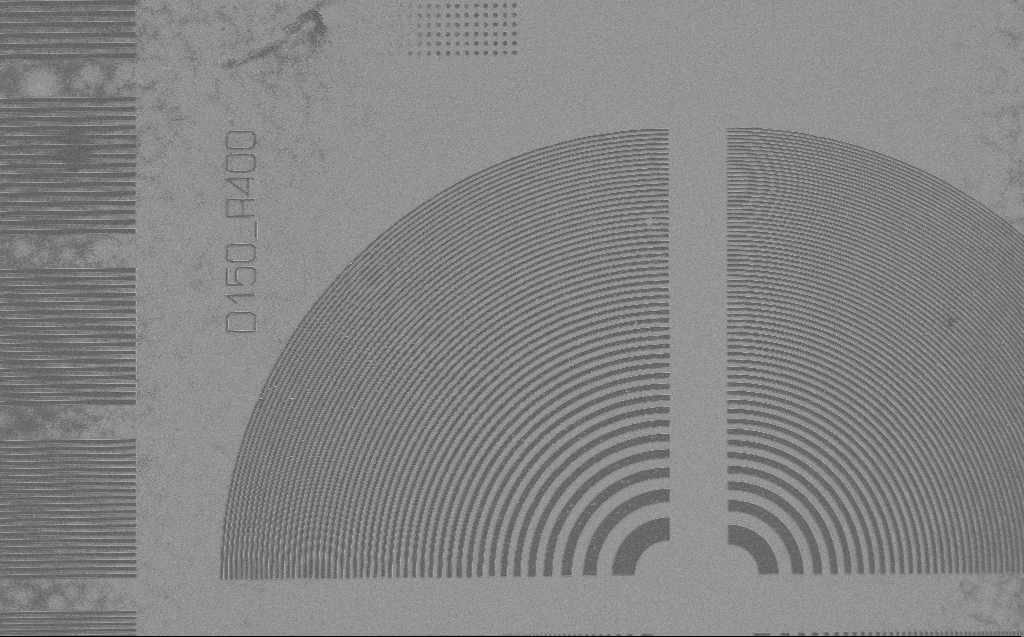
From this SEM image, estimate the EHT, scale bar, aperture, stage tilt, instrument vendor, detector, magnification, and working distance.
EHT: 1.2 kV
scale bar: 20000 nm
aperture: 30 µm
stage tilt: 0°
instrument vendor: Zeiss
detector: SE2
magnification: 2.19 K X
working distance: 5 mm